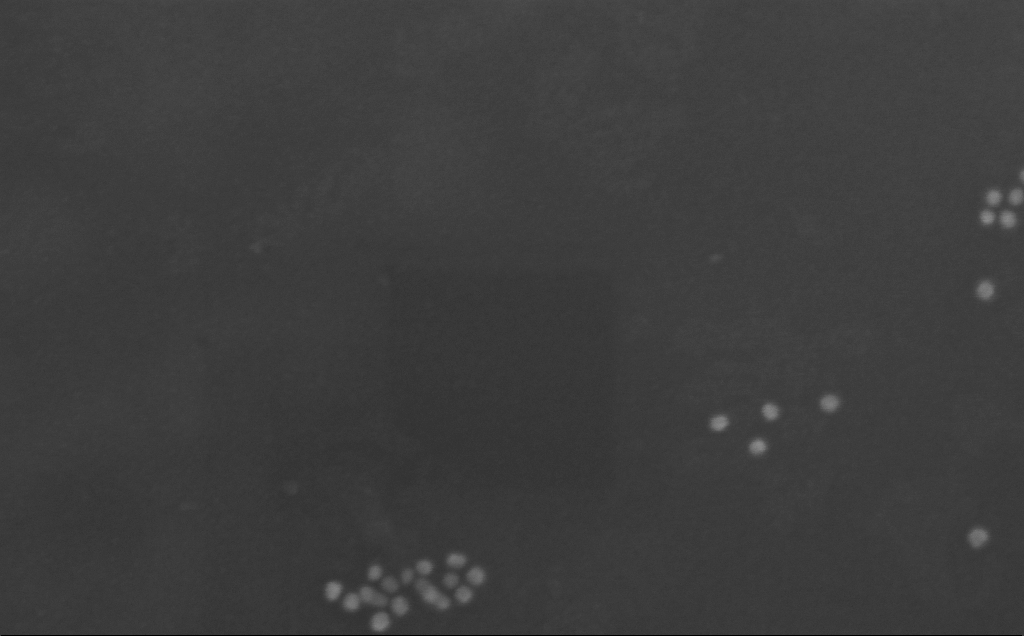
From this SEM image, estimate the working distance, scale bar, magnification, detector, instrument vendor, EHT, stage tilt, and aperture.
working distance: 3 mm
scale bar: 100 nm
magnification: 396.09 K X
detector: InLens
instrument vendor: Zeiss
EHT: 10 kV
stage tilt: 0°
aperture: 30 µm